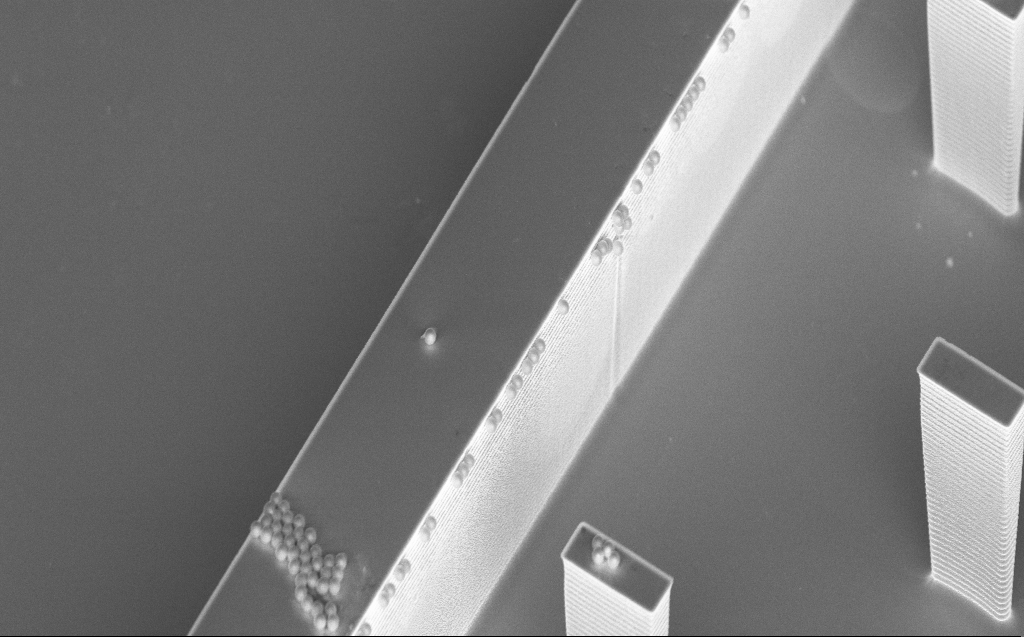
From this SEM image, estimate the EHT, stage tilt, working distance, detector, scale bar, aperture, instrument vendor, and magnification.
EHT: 5 kV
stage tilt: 45°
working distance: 7 mm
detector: InLens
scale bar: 10000 nm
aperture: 30 µm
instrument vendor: Zeiss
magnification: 5.3 K X